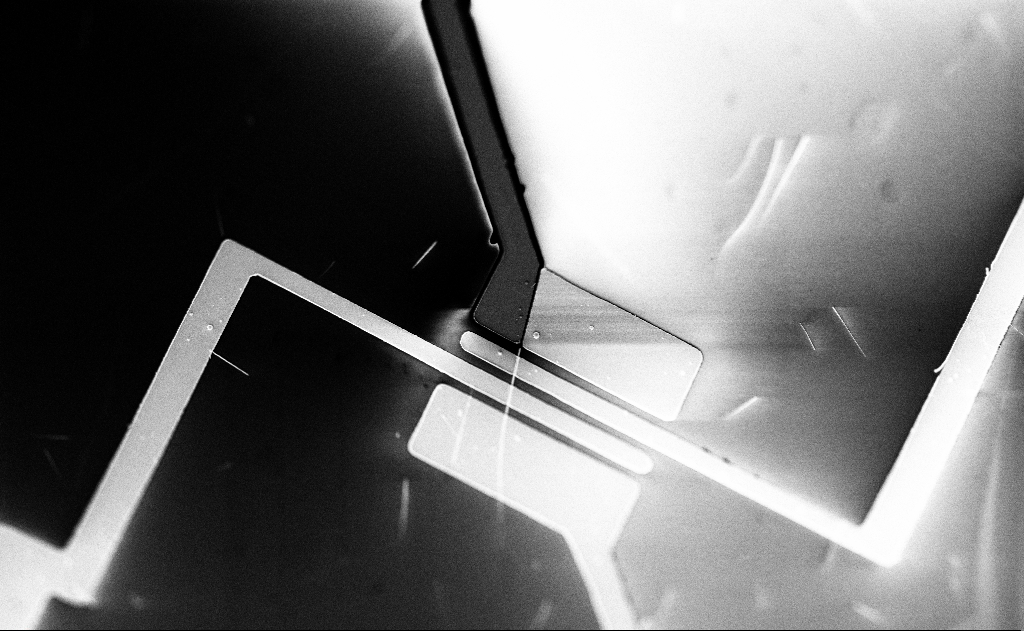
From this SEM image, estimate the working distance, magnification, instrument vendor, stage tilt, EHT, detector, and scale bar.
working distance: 20 mm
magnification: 2.68 K X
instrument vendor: Zeiss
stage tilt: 0°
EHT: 5 kV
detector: SE2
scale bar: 10000 nm